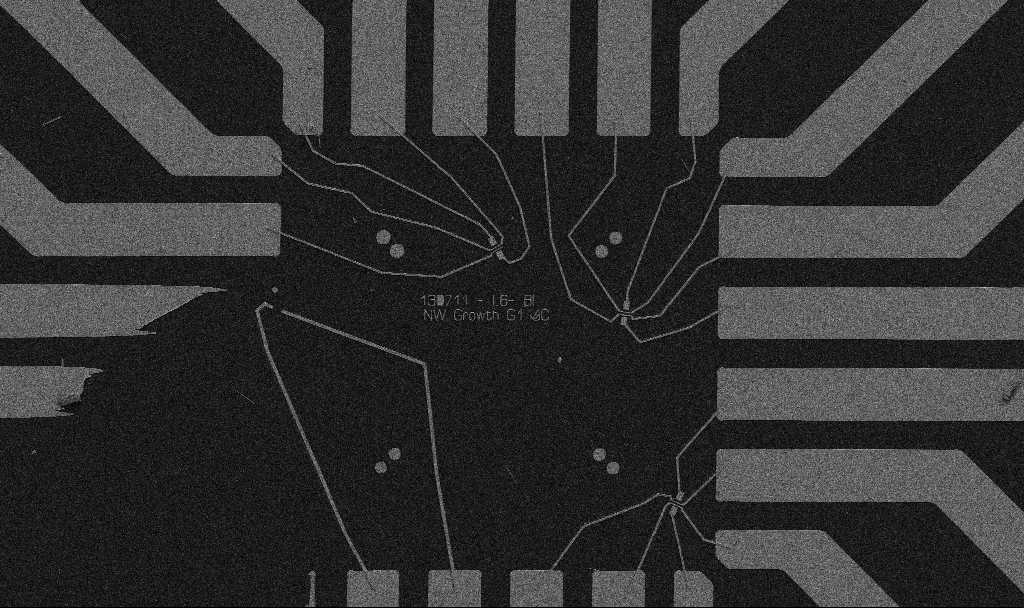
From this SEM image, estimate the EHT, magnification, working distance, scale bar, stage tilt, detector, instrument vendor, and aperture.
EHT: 5 kV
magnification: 1 K X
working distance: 10.7 mm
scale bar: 20000 nm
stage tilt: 0°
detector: SE2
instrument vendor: Zeiss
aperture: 30 µm